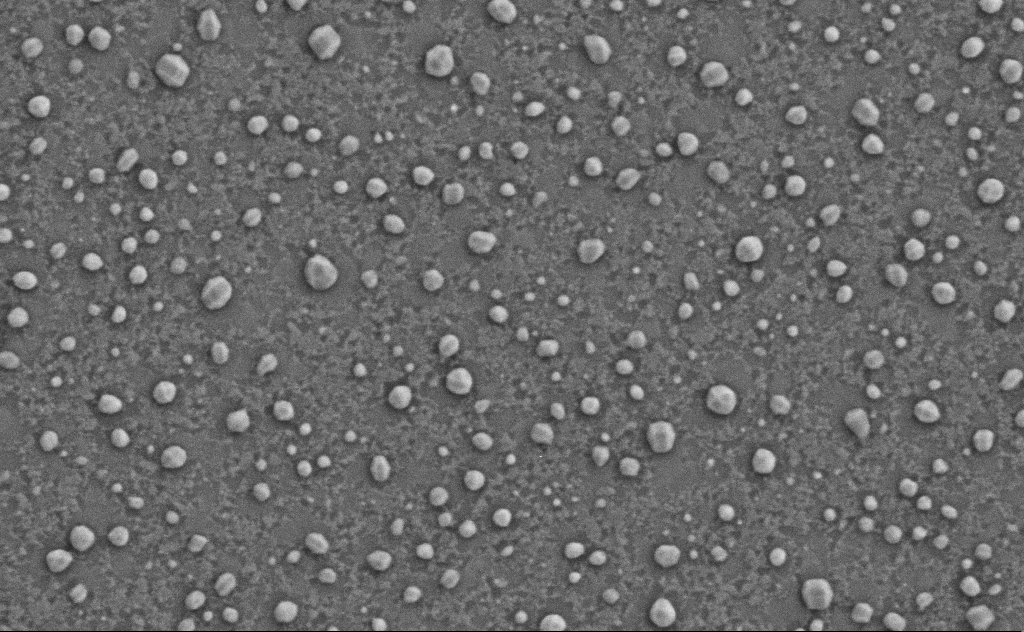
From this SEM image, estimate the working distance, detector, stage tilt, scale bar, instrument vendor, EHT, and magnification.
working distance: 4 mm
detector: SE2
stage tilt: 0°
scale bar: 200 nm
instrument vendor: Zeiss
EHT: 3 kV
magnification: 80 K X